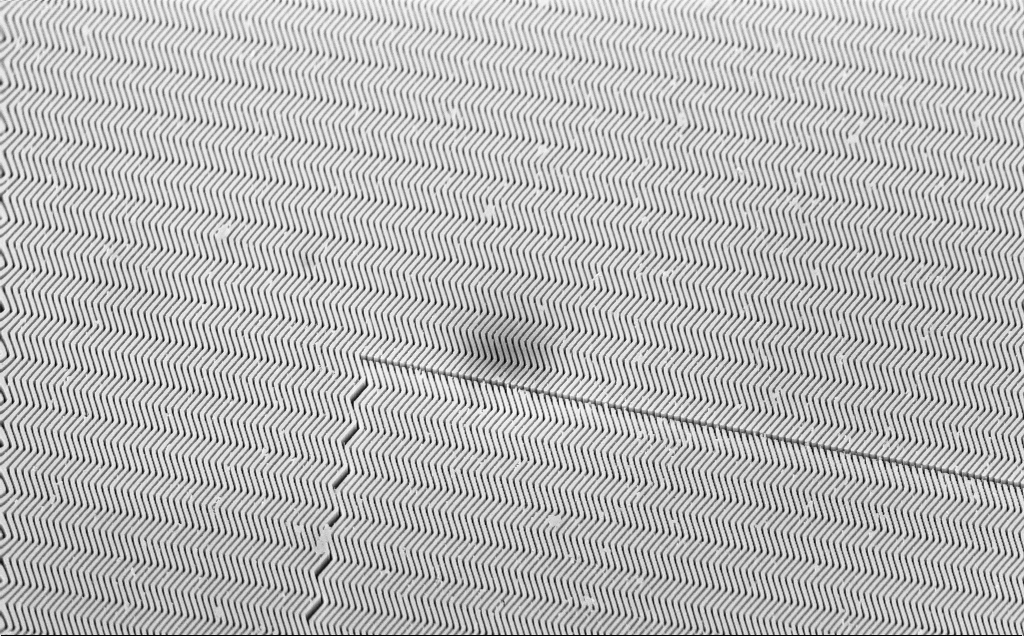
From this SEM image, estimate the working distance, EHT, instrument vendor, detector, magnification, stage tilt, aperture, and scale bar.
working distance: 6 mm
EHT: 10 kV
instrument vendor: Zeiss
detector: InLens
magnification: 8.22 K X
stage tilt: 45°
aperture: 30 µm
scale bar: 2000 nm